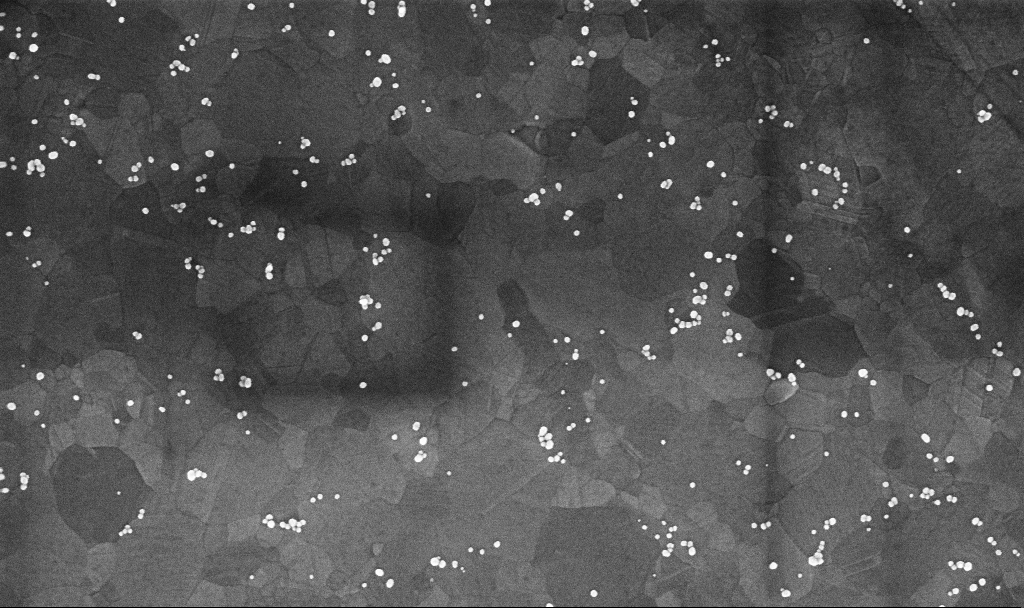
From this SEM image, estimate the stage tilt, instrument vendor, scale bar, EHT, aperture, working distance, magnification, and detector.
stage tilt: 0°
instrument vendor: Zeiss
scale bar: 200 nm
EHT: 10 kV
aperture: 30 µm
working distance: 3.4 mm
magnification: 100.42 K X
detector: InLens